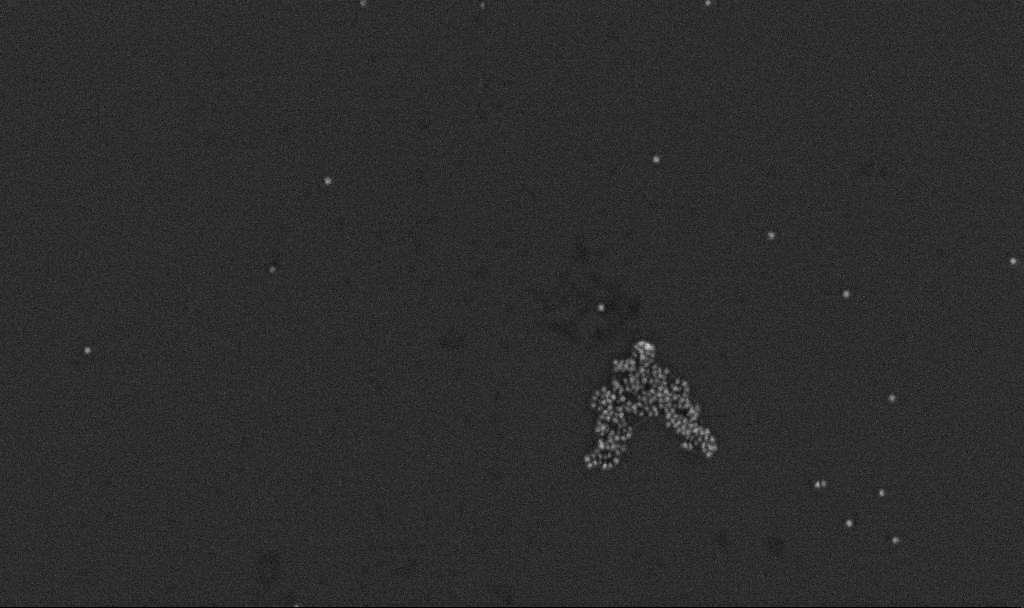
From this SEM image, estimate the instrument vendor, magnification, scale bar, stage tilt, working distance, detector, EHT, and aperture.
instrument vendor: Zeiss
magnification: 100 K X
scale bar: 200 nm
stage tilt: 0°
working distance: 3.3 mm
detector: InLens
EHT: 10 kV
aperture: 30 µm